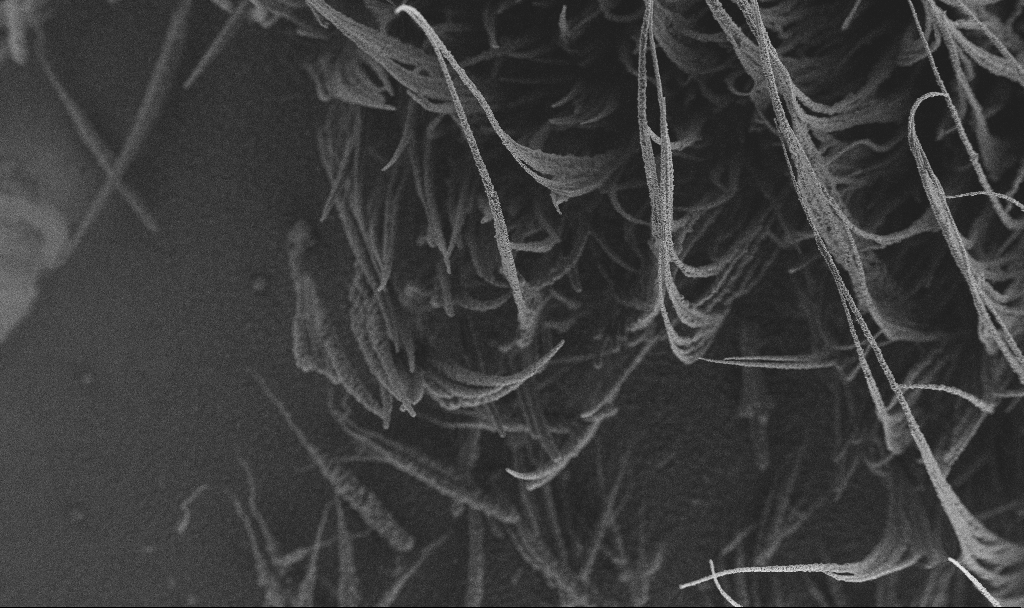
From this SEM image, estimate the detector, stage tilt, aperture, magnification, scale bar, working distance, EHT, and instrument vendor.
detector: SE2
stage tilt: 0°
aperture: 30 µm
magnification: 0.15 K X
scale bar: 100000 nm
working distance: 2.9 mm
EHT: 3 kV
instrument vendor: Zeiss